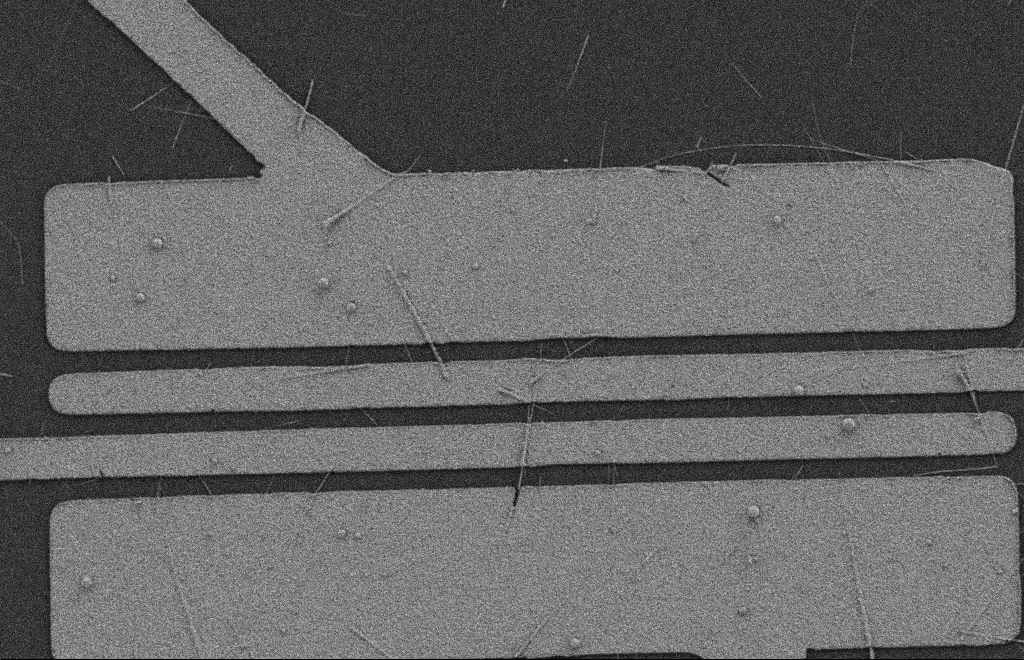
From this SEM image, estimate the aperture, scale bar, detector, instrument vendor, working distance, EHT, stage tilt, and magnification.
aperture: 20 µm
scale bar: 2000 nm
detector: SE2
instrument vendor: Zeiss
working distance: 9 mm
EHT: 2 kV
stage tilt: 0°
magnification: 5.81 K X